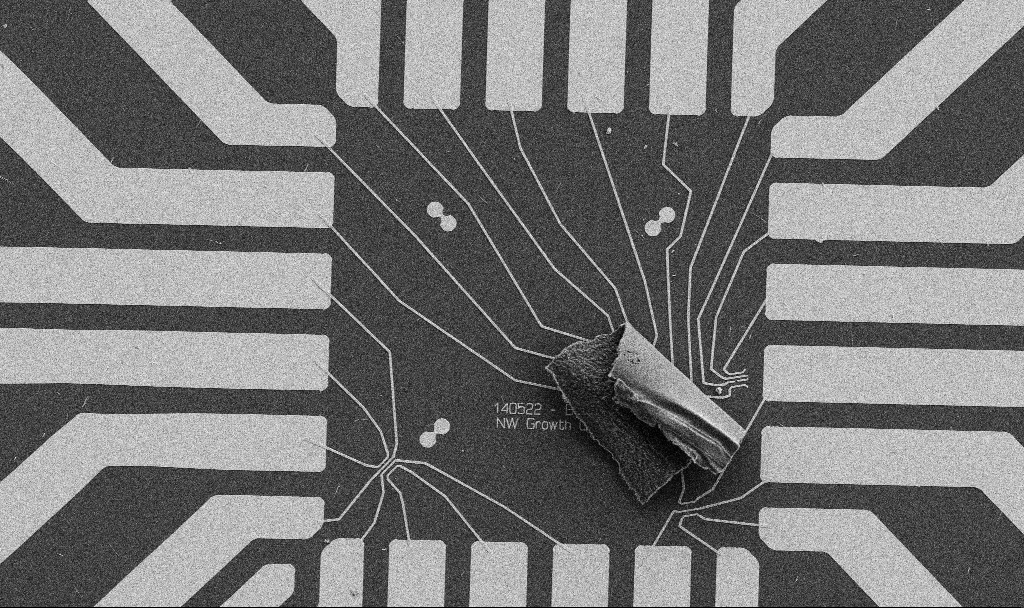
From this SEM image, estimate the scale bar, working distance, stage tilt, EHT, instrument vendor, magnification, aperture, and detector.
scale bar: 20000 nm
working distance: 10.6 mm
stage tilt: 0°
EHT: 5 kV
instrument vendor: Zeiss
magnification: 1 K X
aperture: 30 µm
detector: SE2